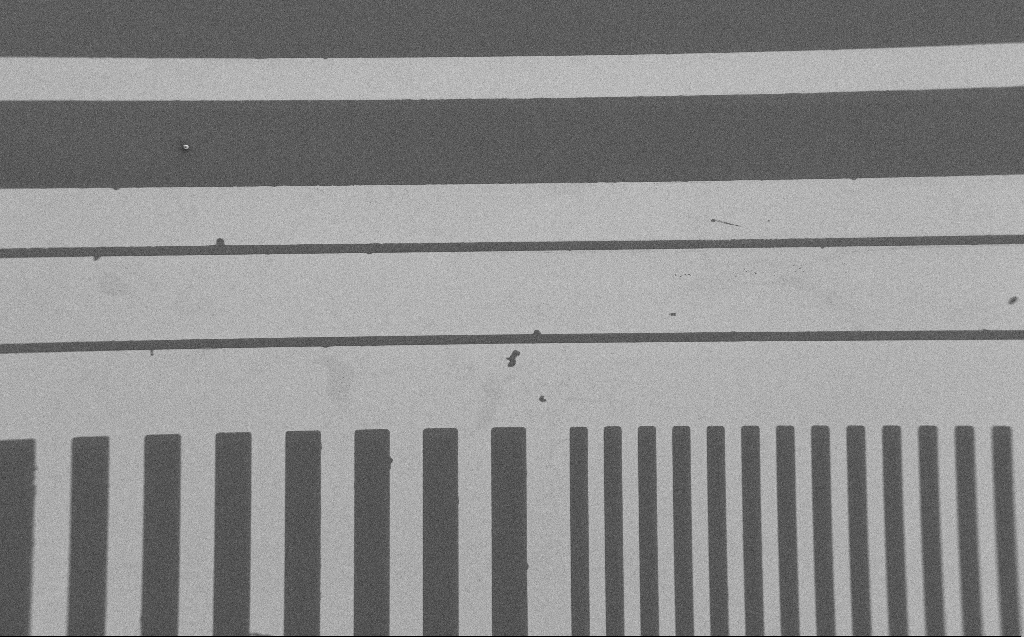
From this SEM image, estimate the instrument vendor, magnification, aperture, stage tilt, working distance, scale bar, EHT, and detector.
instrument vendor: Zeiss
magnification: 0.314 K X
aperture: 30 µm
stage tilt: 0°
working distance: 6 mm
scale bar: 200000 nm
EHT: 1.2 kV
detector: SE2